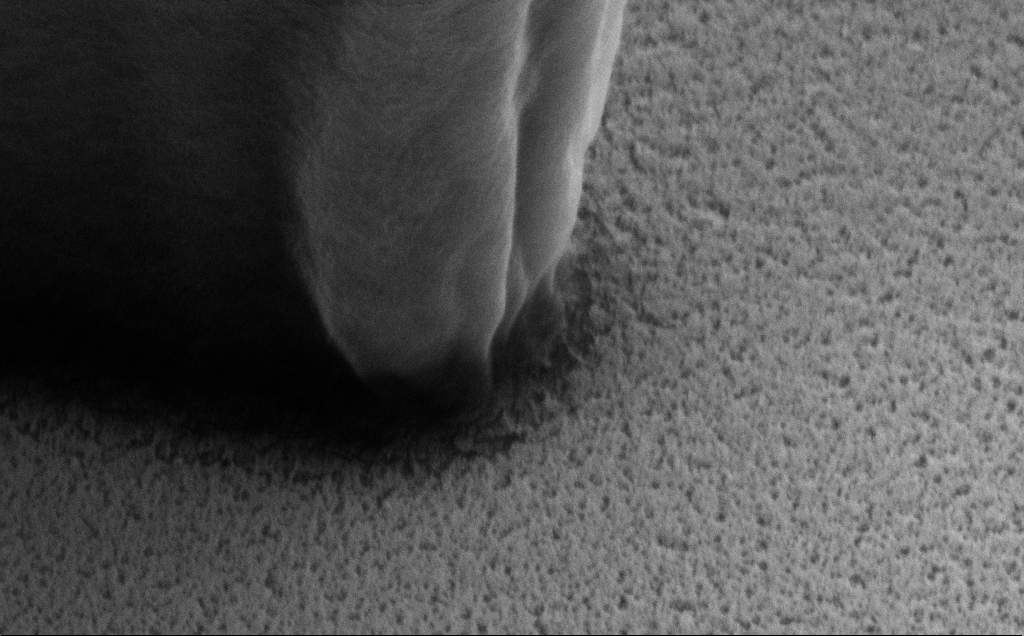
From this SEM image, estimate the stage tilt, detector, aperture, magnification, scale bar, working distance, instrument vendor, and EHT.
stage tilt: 40°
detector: SE2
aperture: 30 µm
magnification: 108.4 K X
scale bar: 200 nm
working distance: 8 mm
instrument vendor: Zeiss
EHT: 5 kV